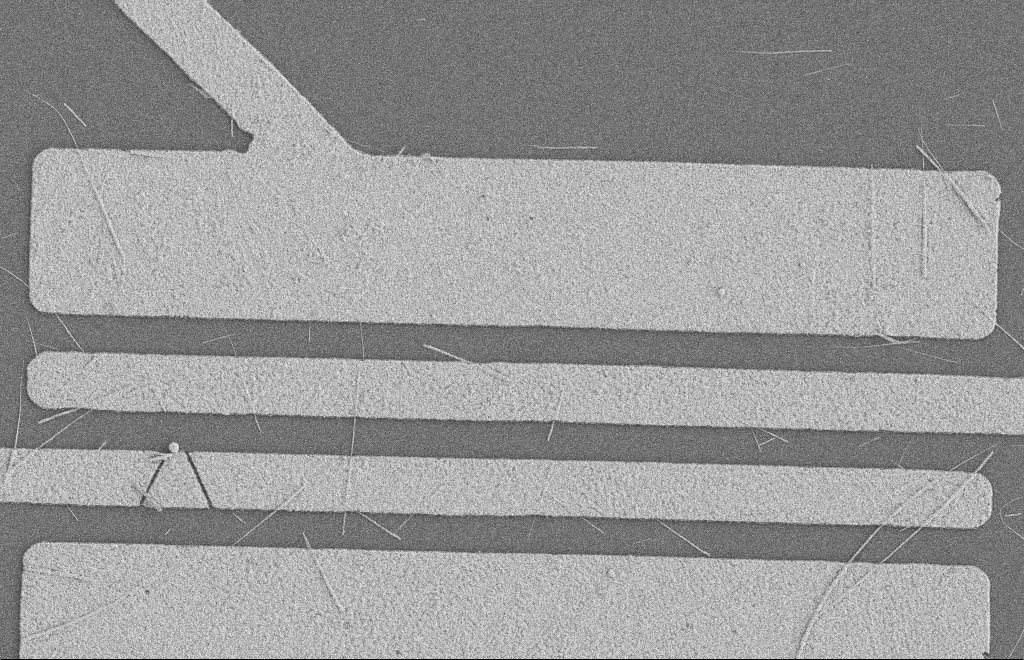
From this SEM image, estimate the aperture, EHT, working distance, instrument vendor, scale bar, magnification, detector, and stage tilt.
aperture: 20 µm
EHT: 2 kV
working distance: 8 mm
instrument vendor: Zeiss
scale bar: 2000 nm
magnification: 5.81 K X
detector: SE2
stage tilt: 0°